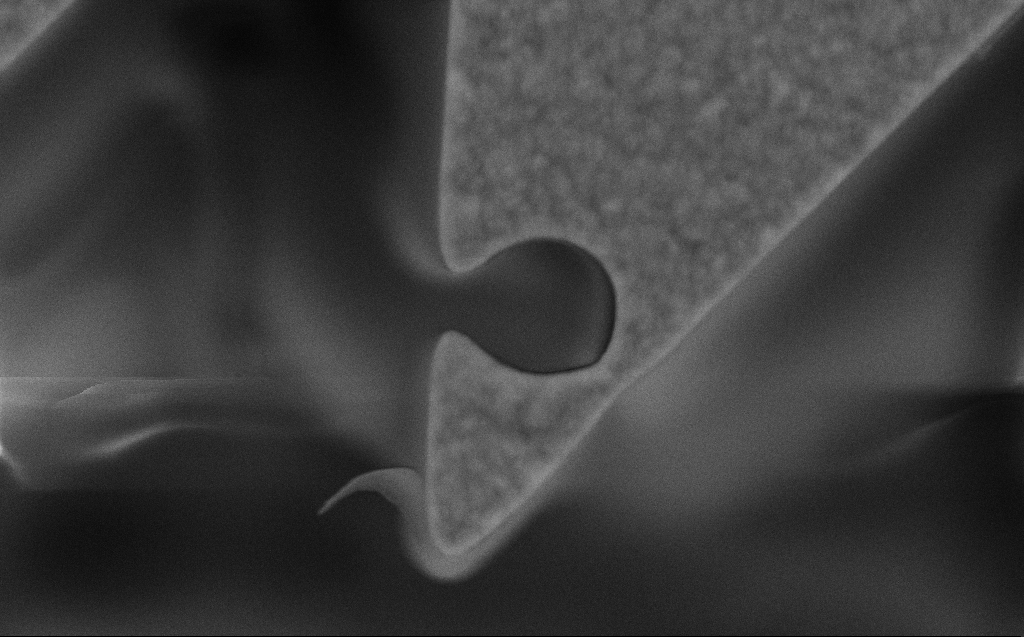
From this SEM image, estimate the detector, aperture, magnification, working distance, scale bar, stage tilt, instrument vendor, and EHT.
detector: SE2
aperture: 30 µm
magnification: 28.61 K X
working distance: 8 mm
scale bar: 2000 nm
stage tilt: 0°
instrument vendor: Zeiss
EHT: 10 kV